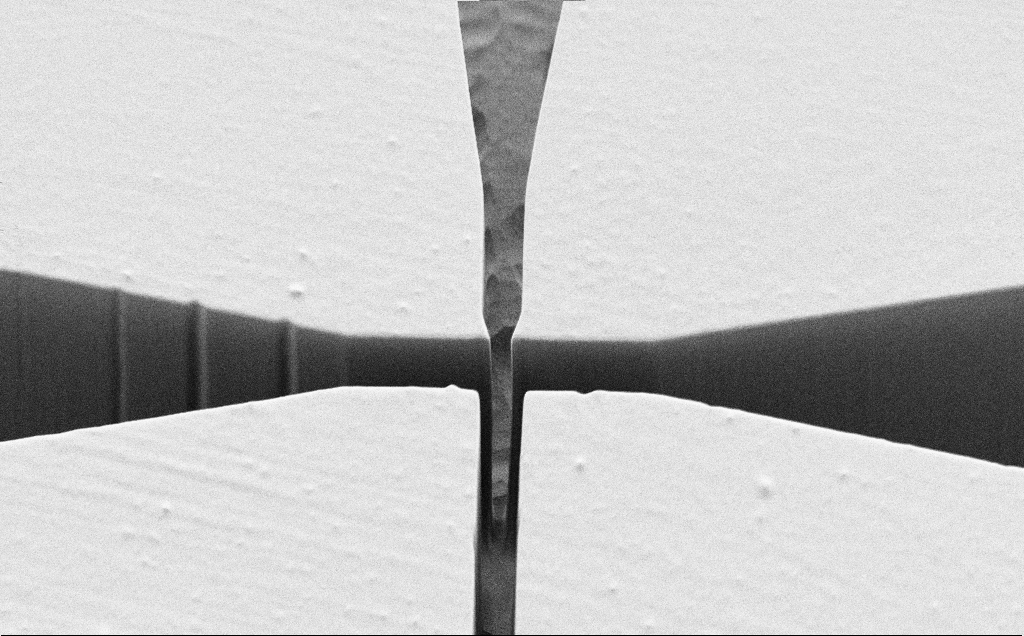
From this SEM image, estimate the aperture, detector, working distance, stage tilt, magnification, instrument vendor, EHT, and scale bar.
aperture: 30 µm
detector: SE2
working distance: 5 mm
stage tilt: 45°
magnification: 1.47 K X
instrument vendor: Zeiss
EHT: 1.2 kV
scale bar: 20000 nm